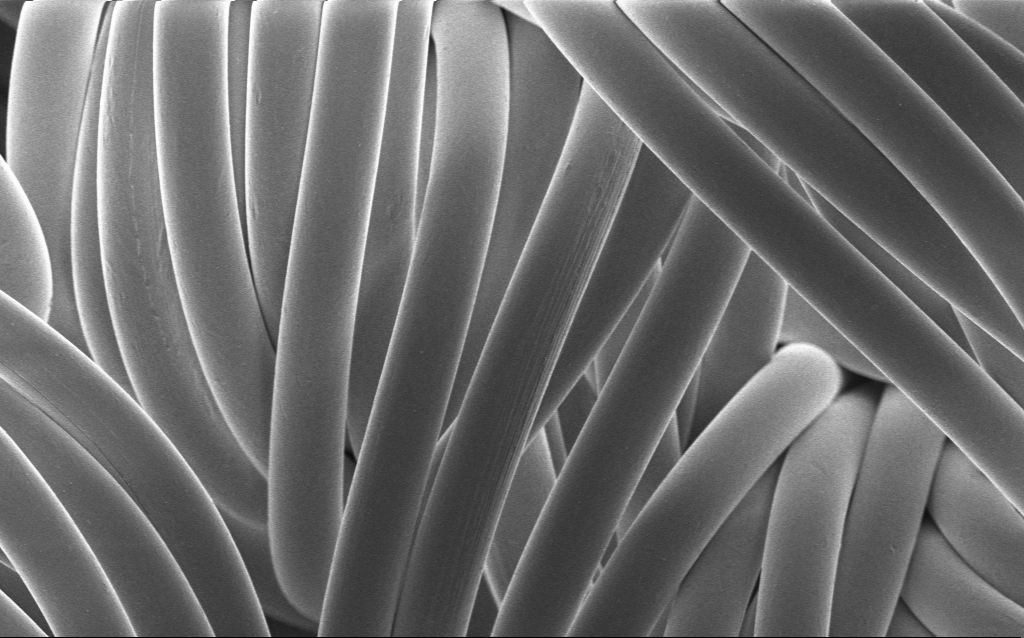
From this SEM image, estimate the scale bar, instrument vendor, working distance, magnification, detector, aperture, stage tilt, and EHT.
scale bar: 20000 nm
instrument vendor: Zeiss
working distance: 4 mm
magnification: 1.14 K X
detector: InLens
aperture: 30 µm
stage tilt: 0°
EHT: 1 kV